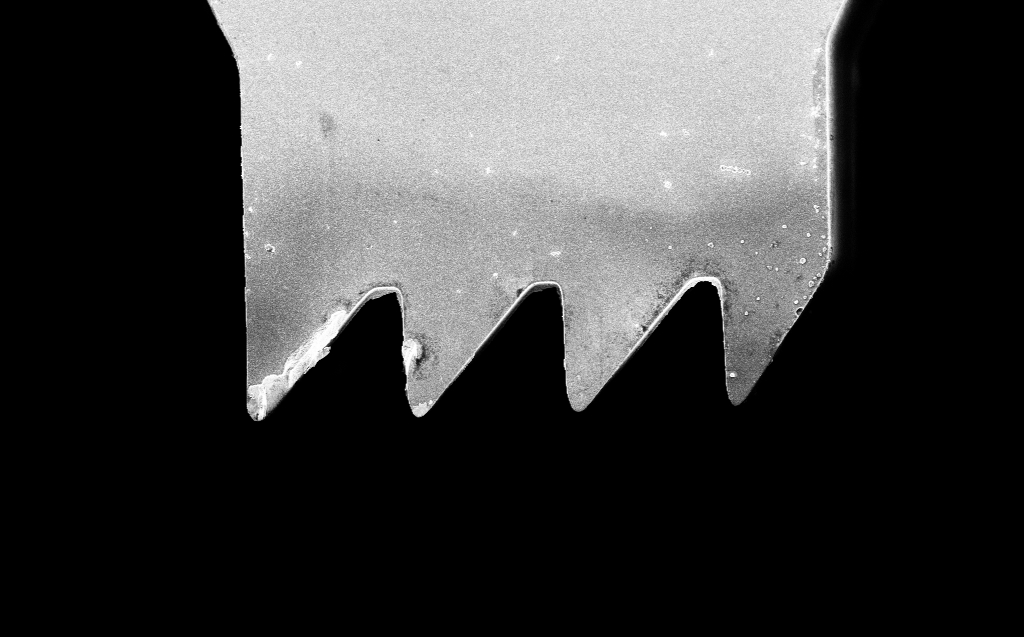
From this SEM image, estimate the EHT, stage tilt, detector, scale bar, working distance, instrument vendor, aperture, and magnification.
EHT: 3 kV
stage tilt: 0°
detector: InLens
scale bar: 10000 nm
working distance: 4 mm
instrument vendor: Zeiss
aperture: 30 µm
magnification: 5.23 K X